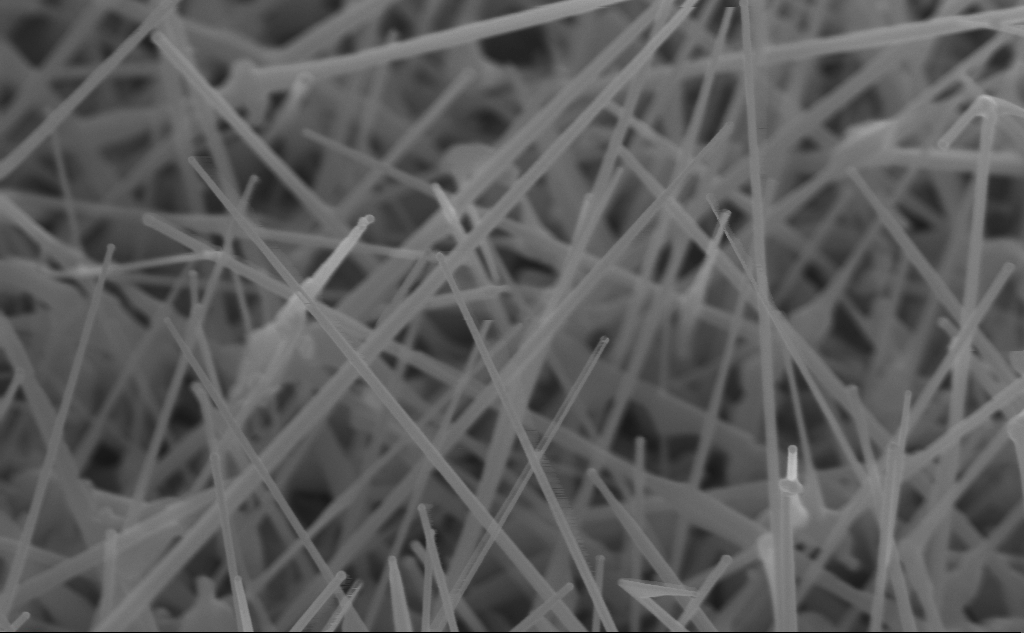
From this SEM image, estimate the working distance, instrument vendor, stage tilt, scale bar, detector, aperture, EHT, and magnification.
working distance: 5 mm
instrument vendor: Zeiss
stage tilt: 45°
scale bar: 200 nm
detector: InLens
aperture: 30 µm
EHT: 10 kV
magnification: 90.42 K X